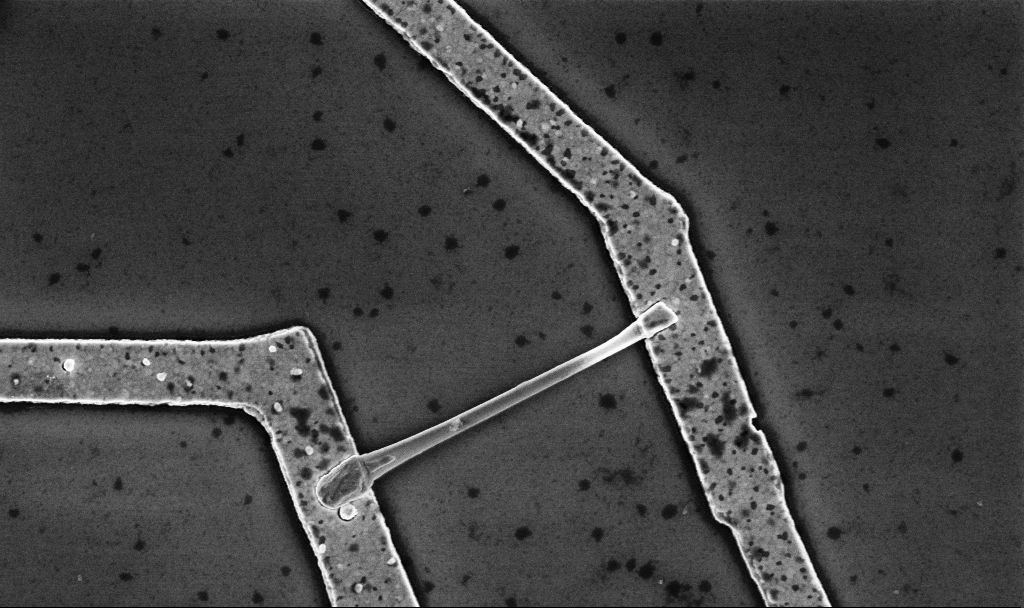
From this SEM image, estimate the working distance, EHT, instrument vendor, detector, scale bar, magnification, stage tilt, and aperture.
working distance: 8.7 mm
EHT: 5 kV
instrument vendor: Zeiss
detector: InLens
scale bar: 1000 nm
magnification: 30 K X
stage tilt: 0°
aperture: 30 µm